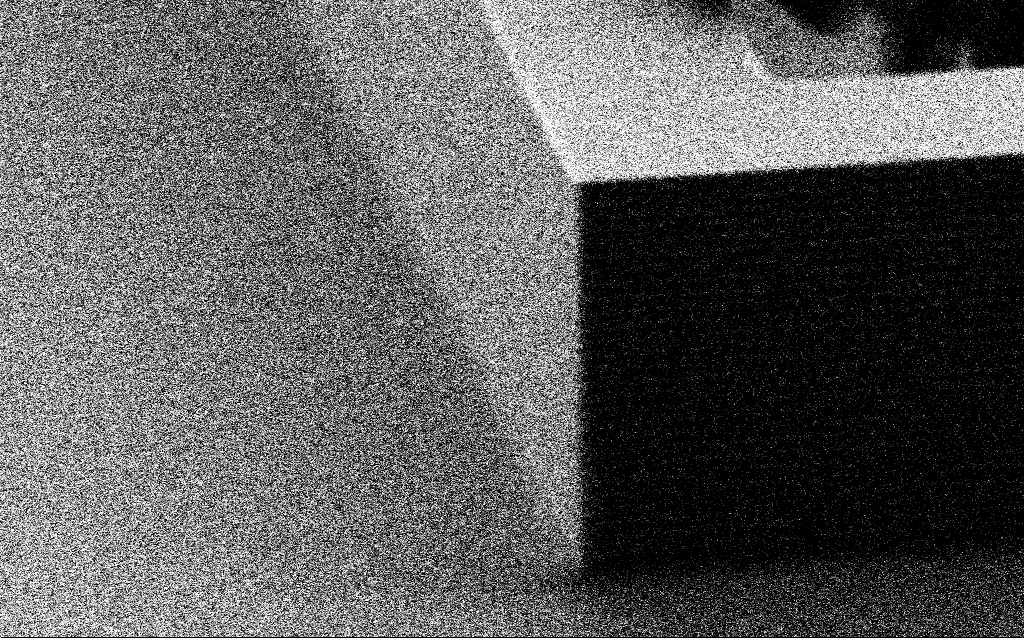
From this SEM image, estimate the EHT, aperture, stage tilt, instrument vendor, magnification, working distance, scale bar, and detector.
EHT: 5 kV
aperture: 30 µm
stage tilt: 70°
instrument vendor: Zeiss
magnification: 17.05 K X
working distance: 4 mm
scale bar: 2000 nm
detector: SE2